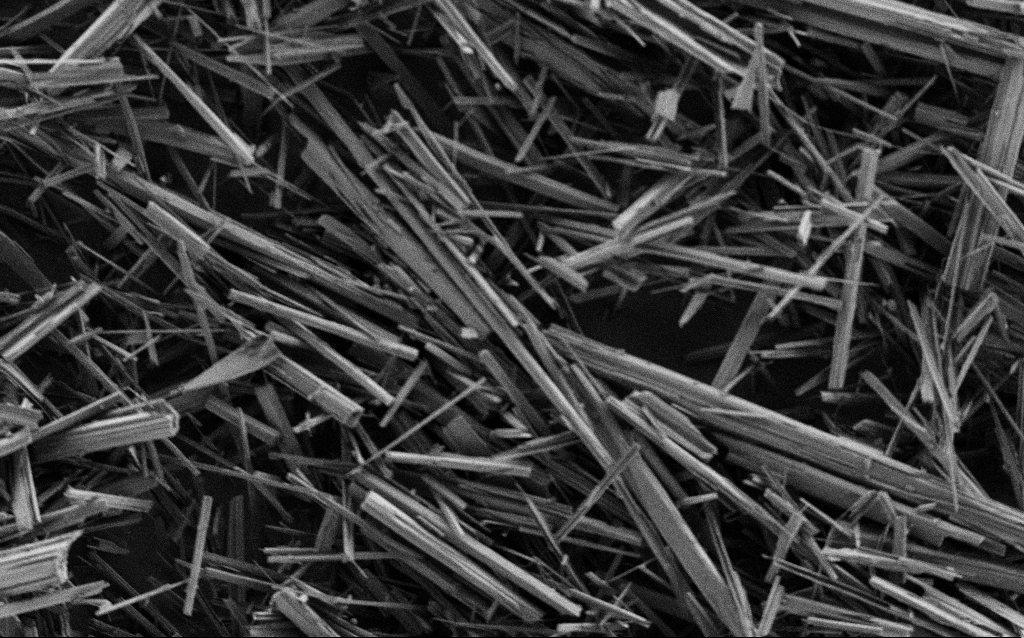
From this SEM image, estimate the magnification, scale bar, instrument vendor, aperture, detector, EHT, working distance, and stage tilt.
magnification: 1 K X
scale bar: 20000 nm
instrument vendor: Zeiss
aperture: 30 µm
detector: SE2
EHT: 0.9 kV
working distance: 4.3 mm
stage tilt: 0°